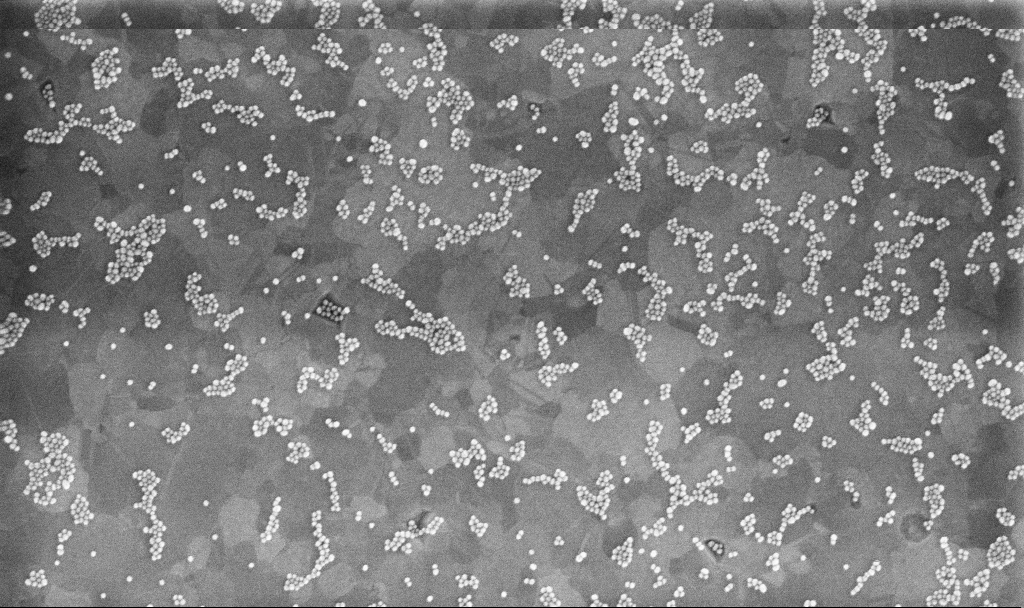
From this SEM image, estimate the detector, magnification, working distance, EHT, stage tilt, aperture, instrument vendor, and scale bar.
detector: InLens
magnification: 100 K X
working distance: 3.3 mm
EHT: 10 kV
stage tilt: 0°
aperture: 30 µm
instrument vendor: Zeiss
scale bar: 200 nm